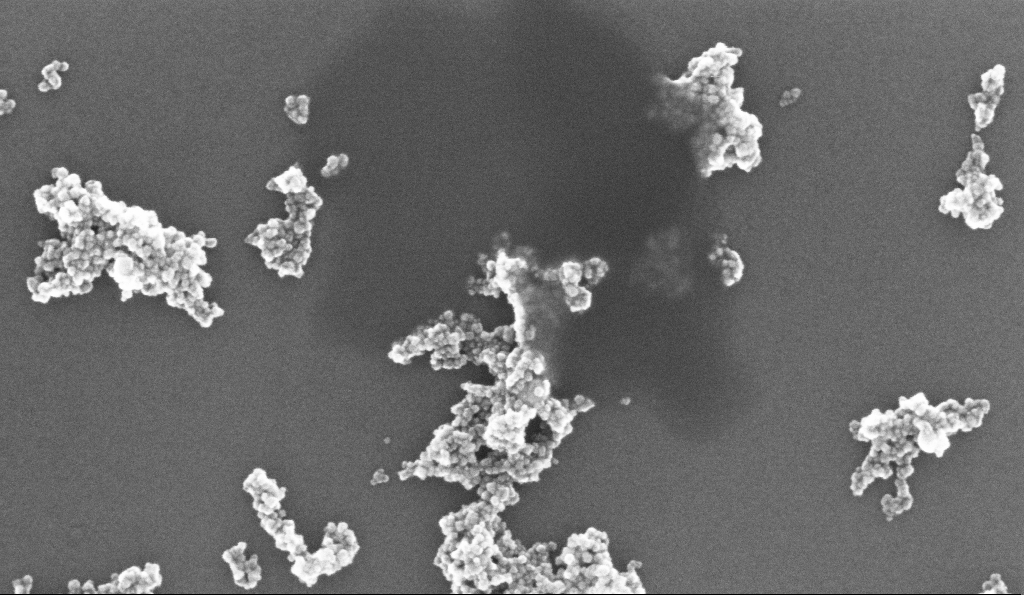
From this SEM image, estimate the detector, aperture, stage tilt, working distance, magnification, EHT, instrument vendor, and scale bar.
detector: InLens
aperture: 30 µm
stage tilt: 0°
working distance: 5.2 mm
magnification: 194.83 K X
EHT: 10 kV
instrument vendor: Zeiss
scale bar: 100 nm